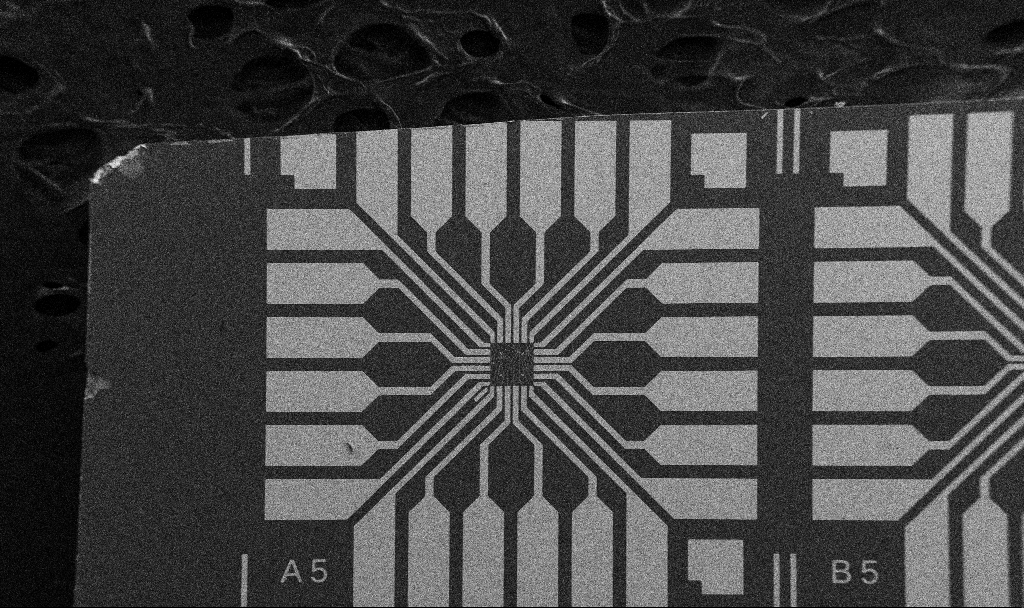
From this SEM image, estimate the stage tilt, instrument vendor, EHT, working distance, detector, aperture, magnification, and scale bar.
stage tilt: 0°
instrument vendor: Zeiss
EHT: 5 kV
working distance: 10.7 mm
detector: SE2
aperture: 30 µm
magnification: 0.1 K X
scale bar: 200000 nm